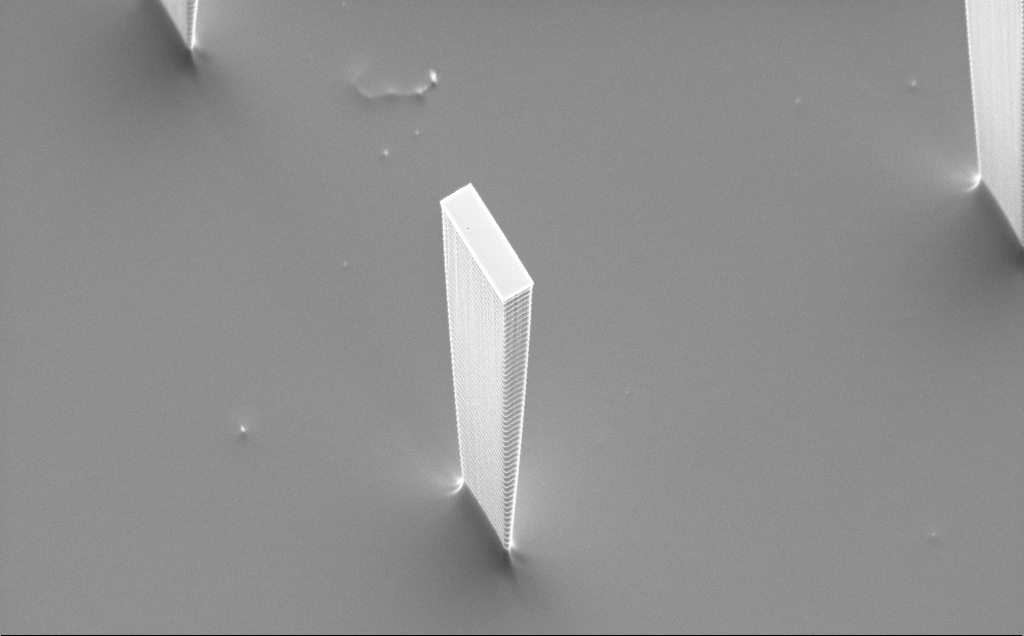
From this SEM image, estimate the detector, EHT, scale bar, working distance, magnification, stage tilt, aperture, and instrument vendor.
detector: SE2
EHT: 5 kV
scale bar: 10000 nm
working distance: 16 mm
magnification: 4.45 K X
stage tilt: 50°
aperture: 30 µm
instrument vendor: Zeiss